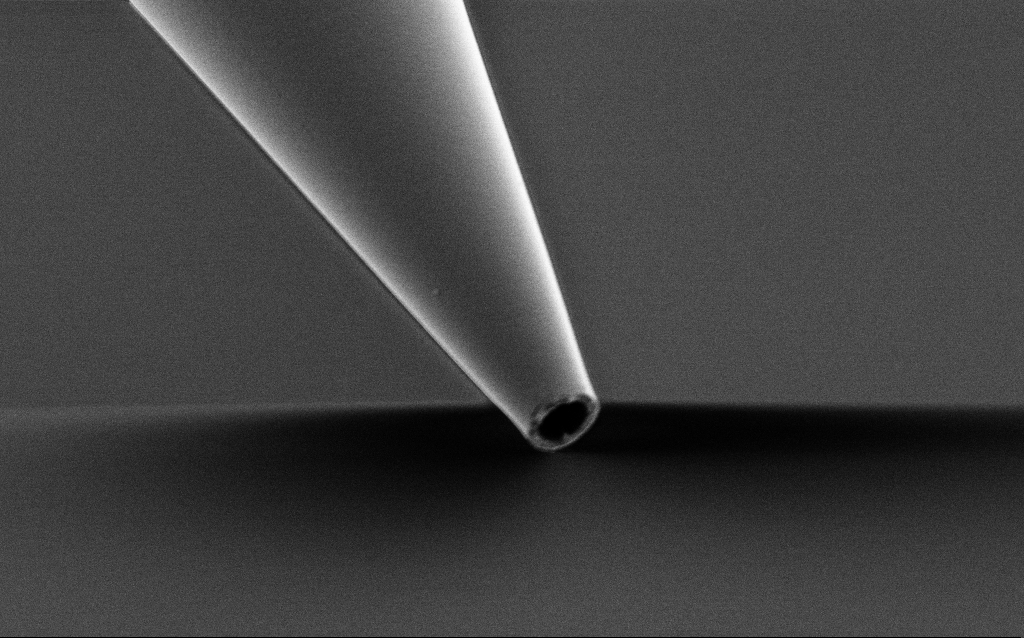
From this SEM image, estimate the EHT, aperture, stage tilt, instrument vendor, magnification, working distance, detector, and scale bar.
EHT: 1 kV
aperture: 30 µm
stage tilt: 45°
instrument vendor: Zeiss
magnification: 10 K X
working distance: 7 mm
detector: SE2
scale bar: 2000 nm